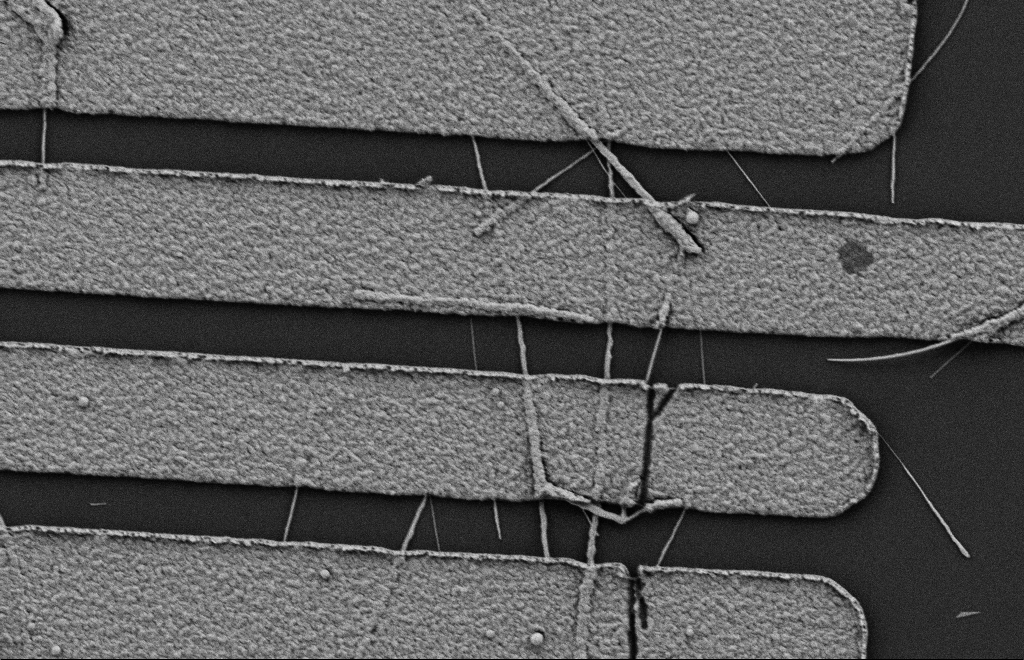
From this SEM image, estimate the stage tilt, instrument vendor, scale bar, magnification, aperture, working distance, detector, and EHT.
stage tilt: -0.3°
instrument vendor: Zeiss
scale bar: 2000 nm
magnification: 16.65 K X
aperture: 20 µm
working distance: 10 mm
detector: SE2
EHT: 2 kV